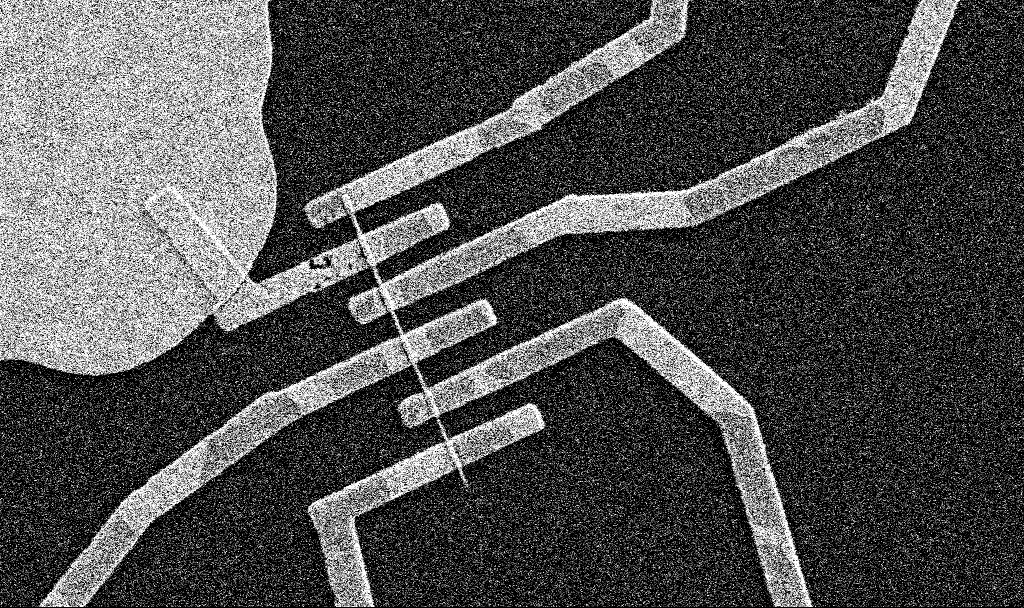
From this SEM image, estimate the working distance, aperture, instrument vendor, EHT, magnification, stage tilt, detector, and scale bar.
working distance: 8.5 mm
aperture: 30 µm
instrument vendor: Zeiss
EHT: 5 kV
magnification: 18.1 K X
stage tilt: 0°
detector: SE2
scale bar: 2000 nm